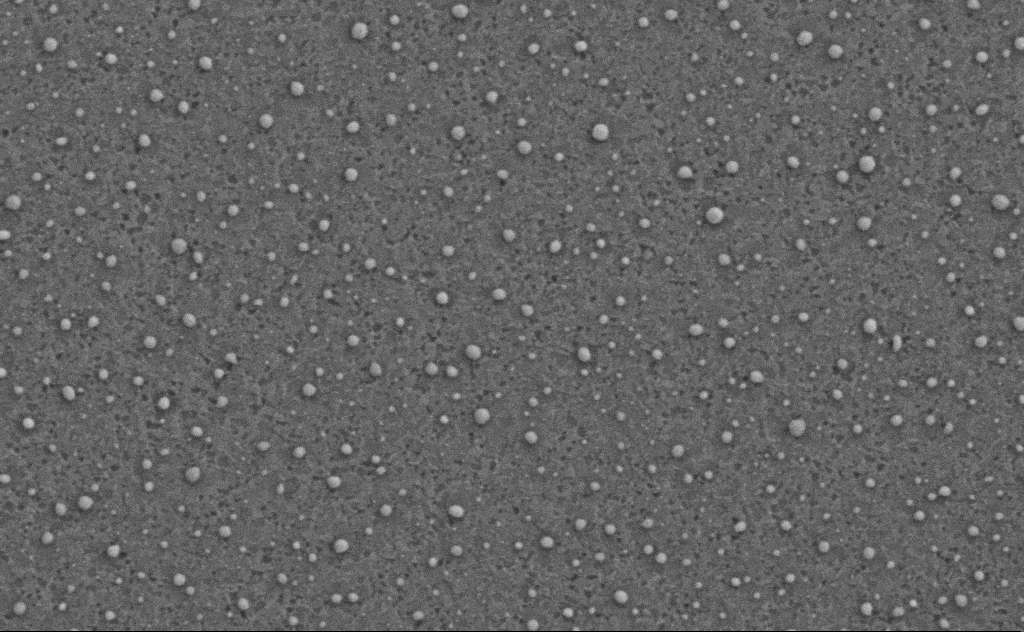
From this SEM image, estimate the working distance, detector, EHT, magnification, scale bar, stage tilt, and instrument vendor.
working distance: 4 mm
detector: SE2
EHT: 3 kV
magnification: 80 K X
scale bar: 200 nm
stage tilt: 0°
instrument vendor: Zeiss